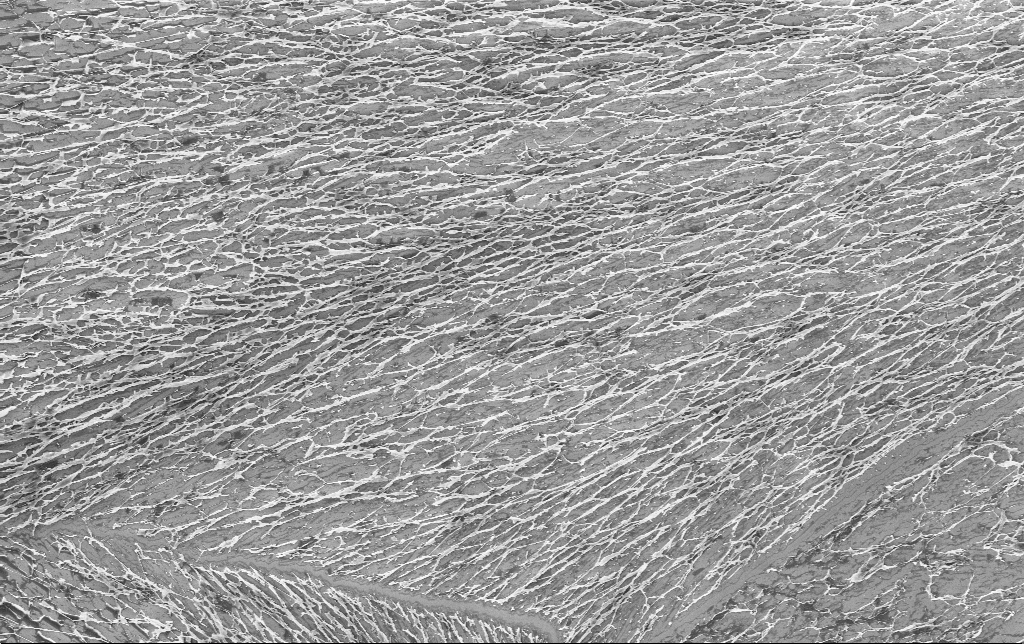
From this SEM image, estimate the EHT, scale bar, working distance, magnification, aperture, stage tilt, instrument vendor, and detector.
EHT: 3 kV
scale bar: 10000 nm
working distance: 3.4 mm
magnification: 2.82 K X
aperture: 30 µm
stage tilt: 0°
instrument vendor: Zeiss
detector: InLens